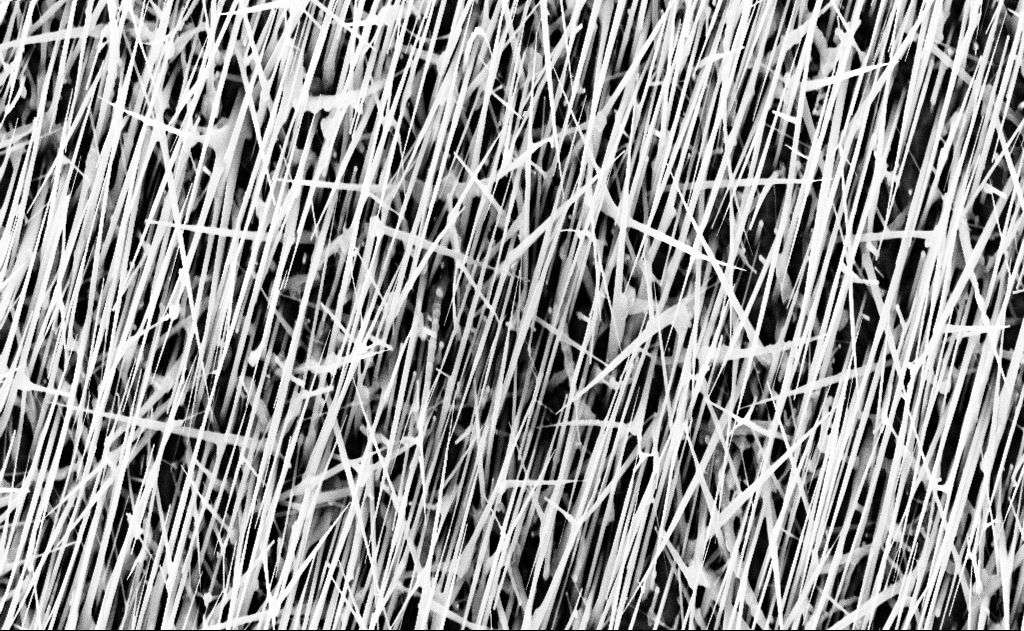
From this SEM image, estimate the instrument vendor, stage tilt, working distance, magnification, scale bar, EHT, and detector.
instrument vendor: Zeiss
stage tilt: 0°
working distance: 16 mm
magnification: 20 K X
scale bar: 2000 nm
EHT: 10 kV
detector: InLens